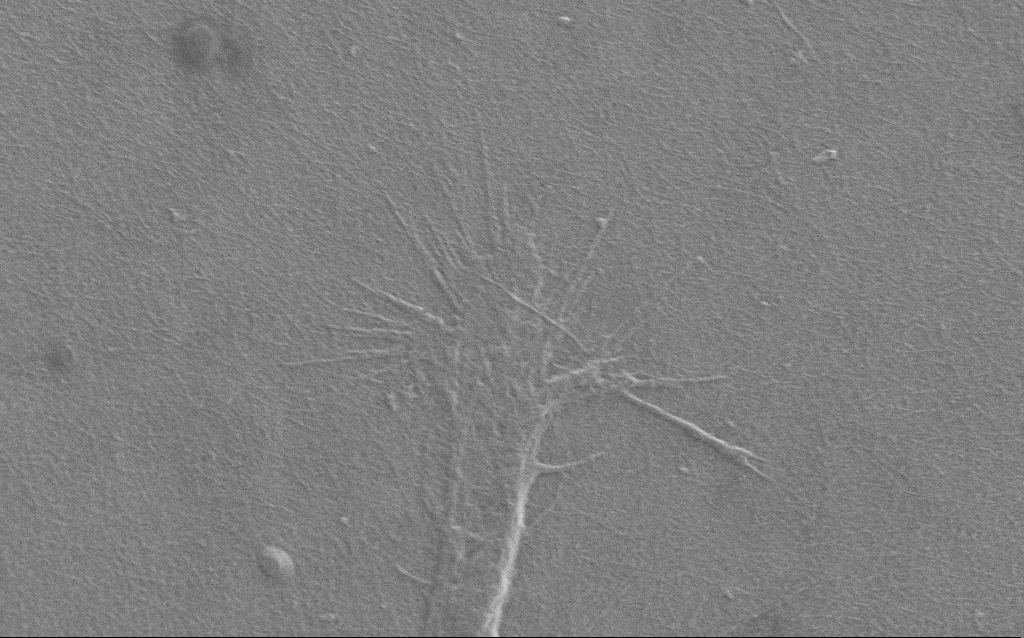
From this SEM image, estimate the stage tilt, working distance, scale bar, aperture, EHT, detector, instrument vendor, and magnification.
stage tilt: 0°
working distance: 6 mm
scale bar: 10000 nm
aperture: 30 µm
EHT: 1 kV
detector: SE2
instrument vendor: Zeiss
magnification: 6.71 K X